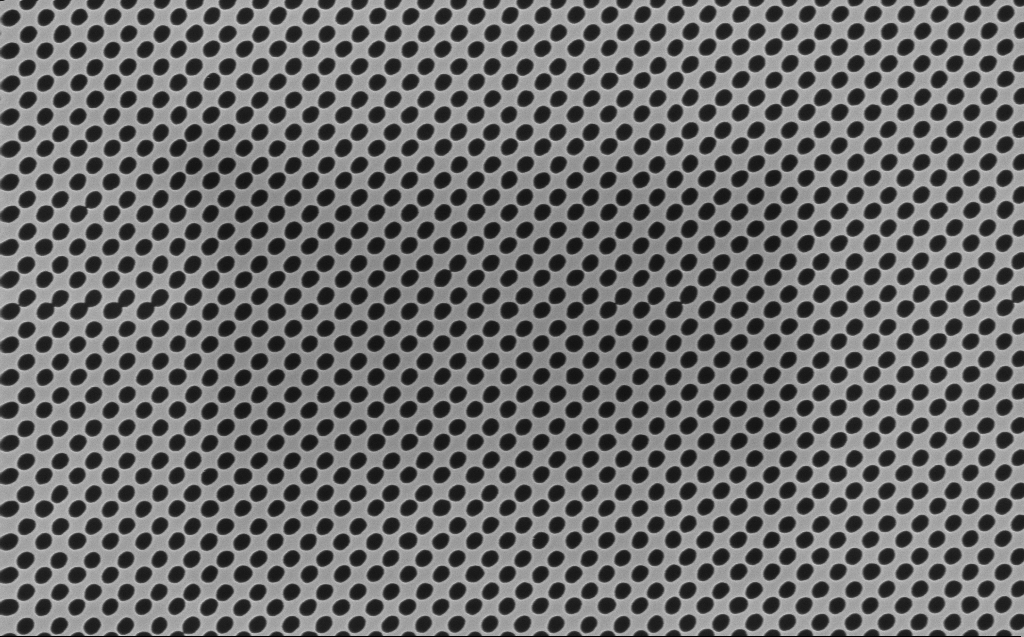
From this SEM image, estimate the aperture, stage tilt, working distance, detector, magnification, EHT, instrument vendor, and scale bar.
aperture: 30 µm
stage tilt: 0°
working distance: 7 mm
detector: InLens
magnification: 40.46 K X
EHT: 5 kV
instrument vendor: Zeiss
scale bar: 1000 nm